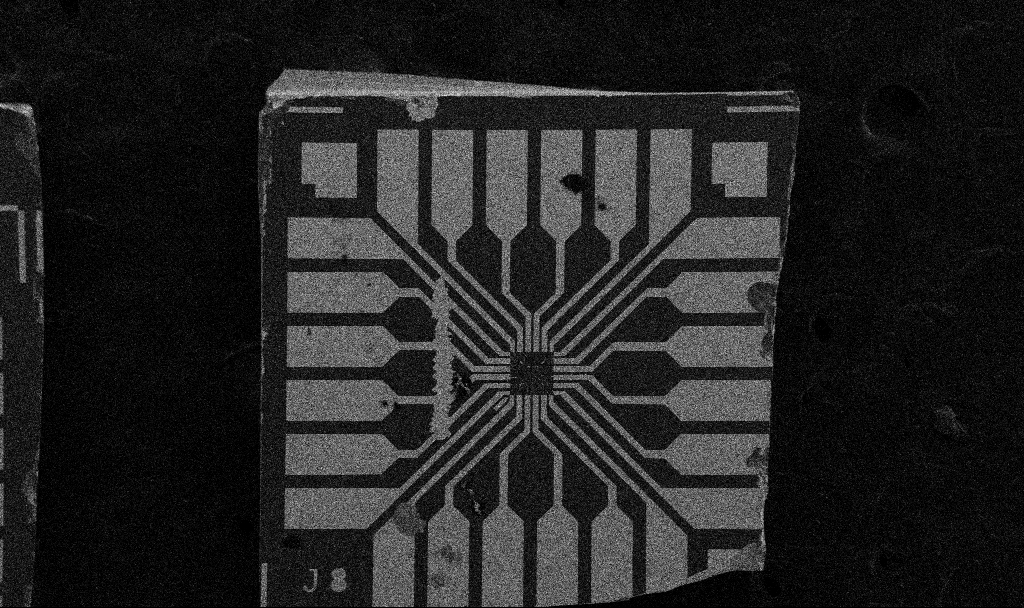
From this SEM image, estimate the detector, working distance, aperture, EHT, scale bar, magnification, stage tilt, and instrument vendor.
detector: SE2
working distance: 10.7 mm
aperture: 30 µm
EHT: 5 kV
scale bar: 200000 nm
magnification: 0.1 K X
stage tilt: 0°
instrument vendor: Zeiss